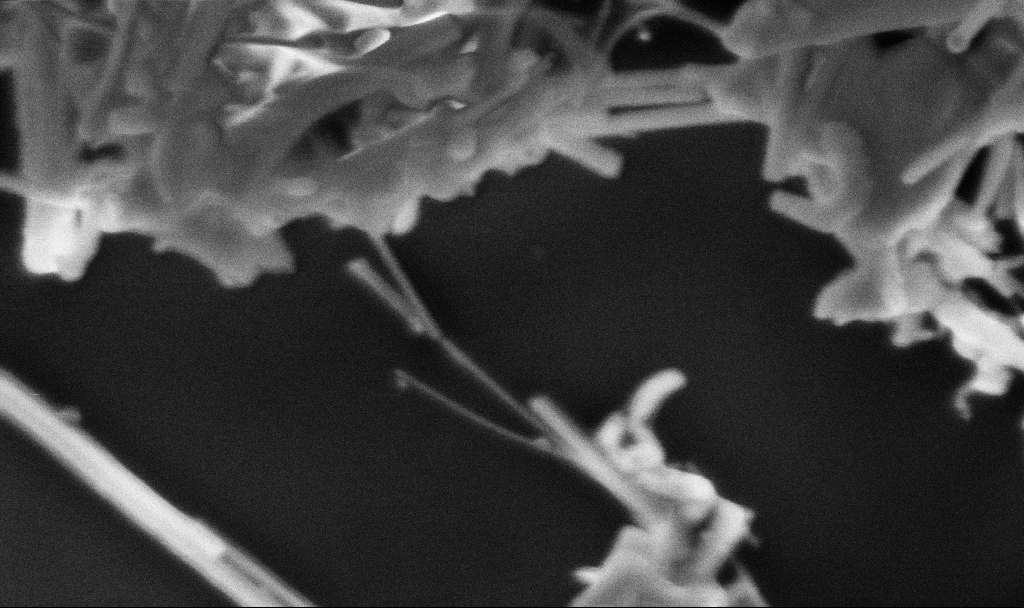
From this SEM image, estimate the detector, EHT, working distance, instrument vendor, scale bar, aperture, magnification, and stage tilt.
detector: InLens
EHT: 3 kV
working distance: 3.3 mm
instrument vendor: Zeiss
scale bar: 200 nm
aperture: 30 µm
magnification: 163.95 K X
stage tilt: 0°